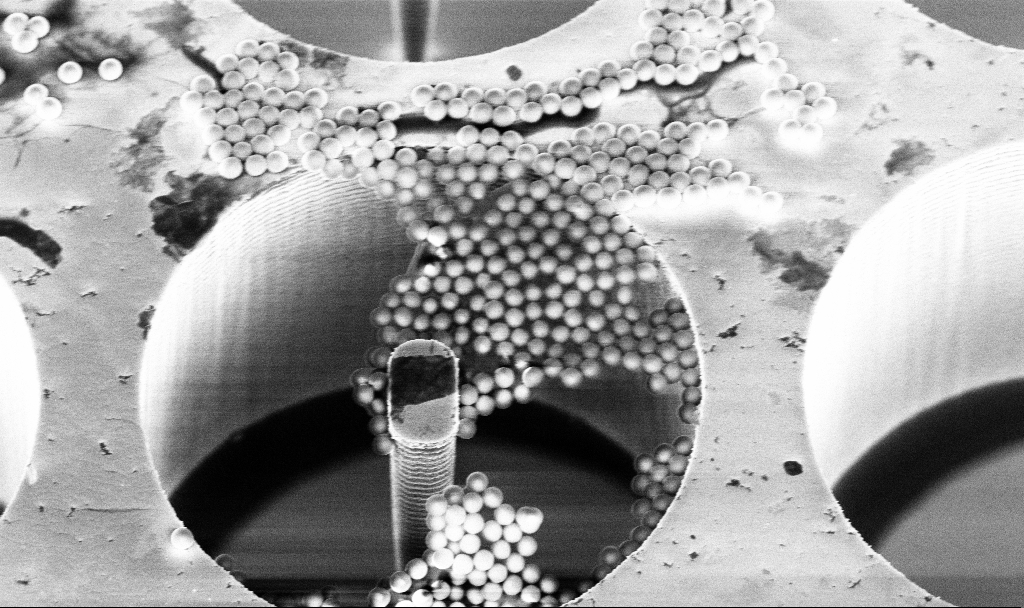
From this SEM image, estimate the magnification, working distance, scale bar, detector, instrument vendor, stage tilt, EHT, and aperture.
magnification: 10.58 K X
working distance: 7.1 mm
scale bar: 2000 nm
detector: InLens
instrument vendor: Zeiss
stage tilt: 30°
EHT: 5 kV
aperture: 30 µm